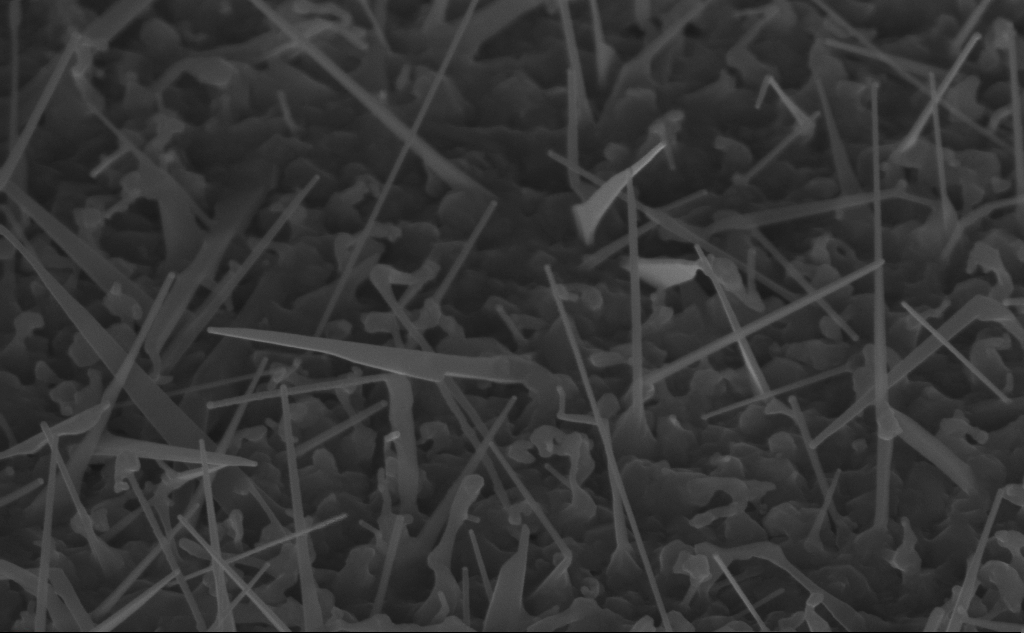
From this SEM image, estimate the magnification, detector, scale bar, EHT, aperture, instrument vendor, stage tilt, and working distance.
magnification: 80 K X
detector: InLens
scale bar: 200 nm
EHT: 10 kV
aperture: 30 µm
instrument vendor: Zeiss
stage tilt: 45°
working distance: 8 mm